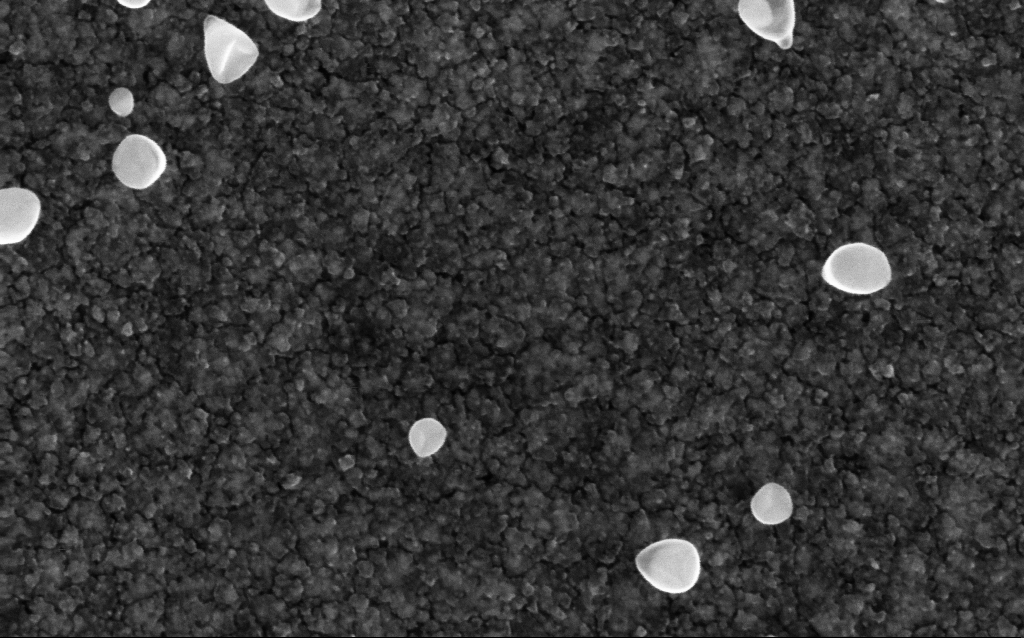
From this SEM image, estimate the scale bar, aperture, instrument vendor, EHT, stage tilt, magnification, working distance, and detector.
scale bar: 100 nm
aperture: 30 µm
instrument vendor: Zeiss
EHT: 5 kV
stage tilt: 0°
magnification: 200 K X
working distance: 1.9 mm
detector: InLens